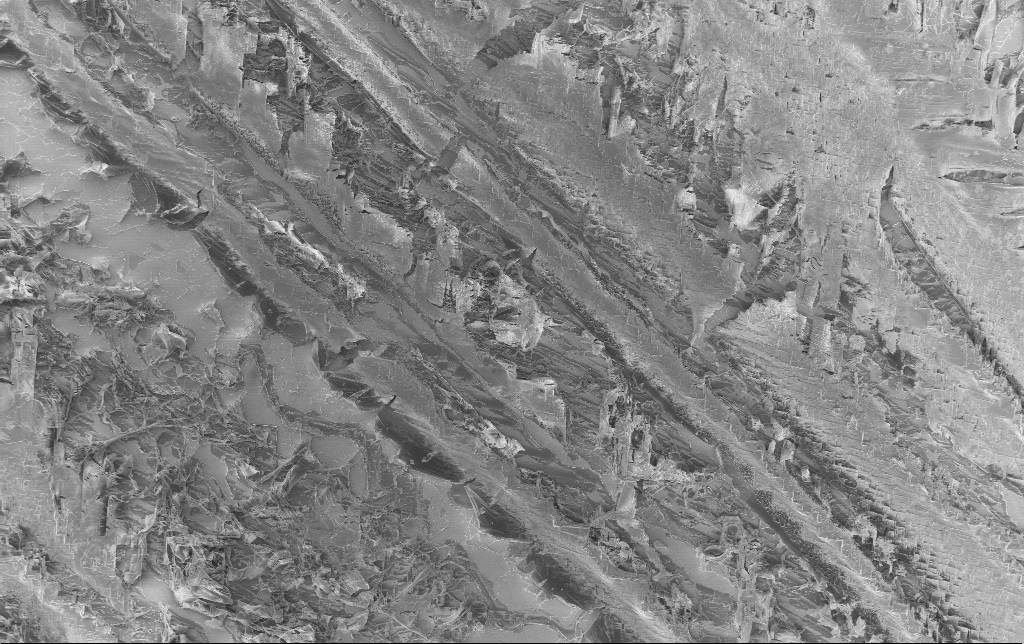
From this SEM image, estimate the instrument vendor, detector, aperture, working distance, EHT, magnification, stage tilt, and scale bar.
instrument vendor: Zeiss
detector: InLens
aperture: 30 µm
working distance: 3.2 mm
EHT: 1.5 kV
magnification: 0.856 K X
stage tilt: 0°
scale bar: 20000 nm